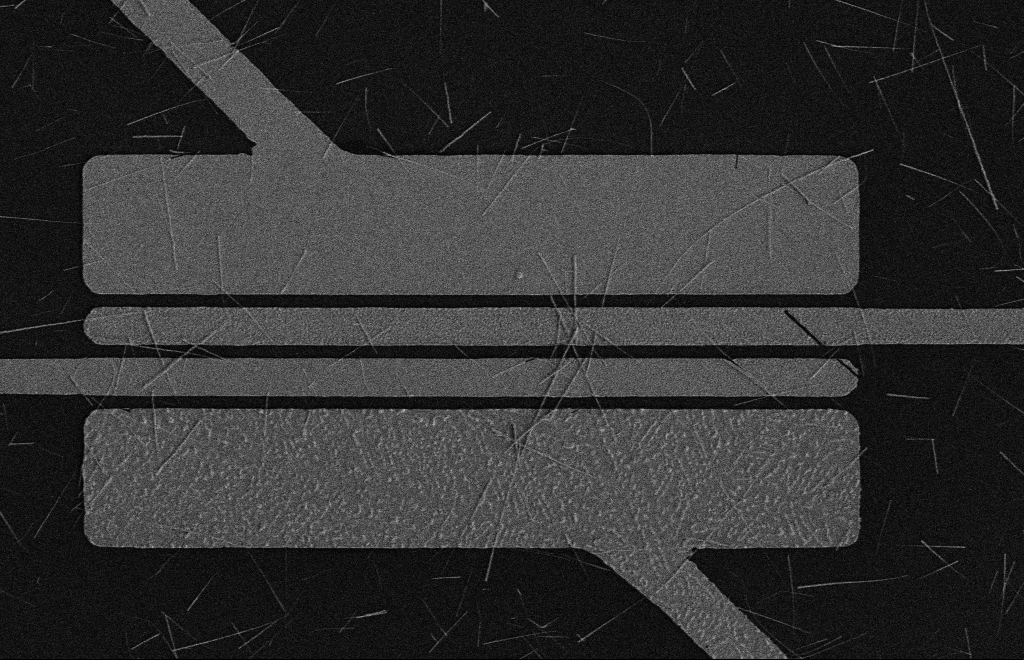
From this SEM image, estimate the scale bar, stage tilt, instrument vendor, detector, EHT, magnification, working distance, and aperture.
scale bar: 10000 nm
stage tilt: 0°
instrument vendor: Zeiss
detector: SE2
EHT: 5 kV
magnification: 4.67 K X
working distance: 16 mm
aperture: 10 µm